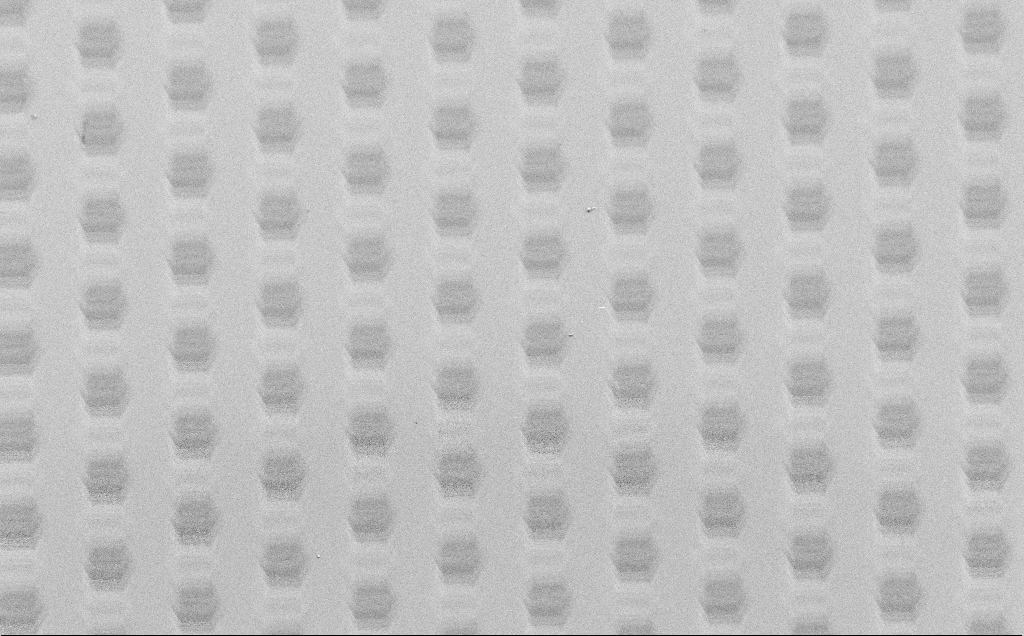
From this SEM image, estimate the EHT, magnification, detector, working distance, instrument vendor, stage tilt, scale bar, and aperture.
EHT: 1.5 kV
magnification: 0.932 K X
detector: SE2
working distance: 6 mm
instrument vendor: Zeiss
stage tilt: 30°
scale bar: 20000 nm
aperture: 30 µm